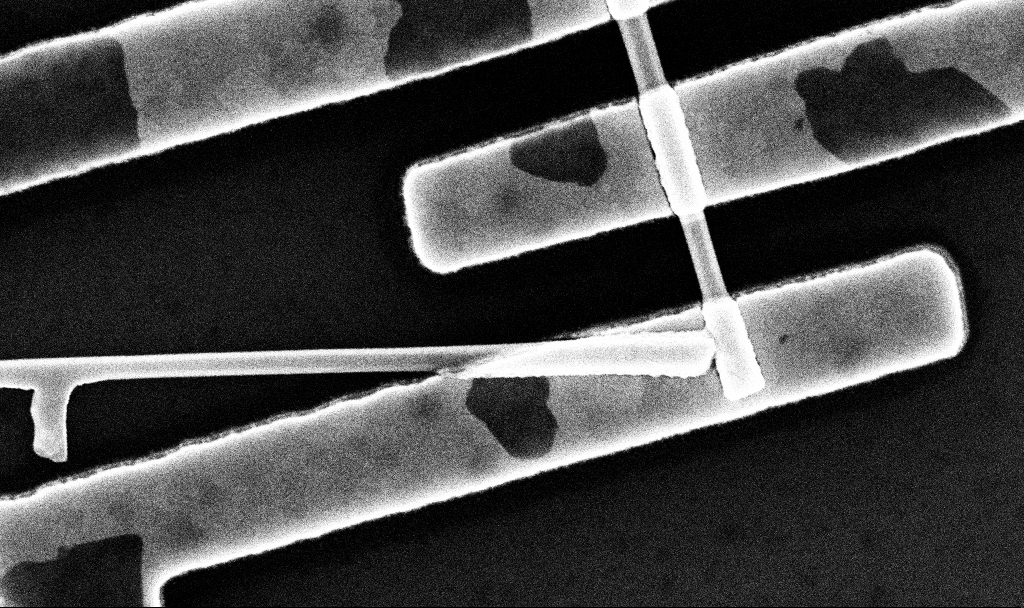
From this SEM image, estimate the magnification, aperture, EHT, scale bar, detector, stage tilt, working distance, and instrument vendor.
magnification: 72.43 K X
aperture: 30 µm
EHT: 10 kV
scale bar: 1000 nm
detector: InLens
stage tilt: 0°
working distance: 6.7 mm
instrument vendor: Zeiss